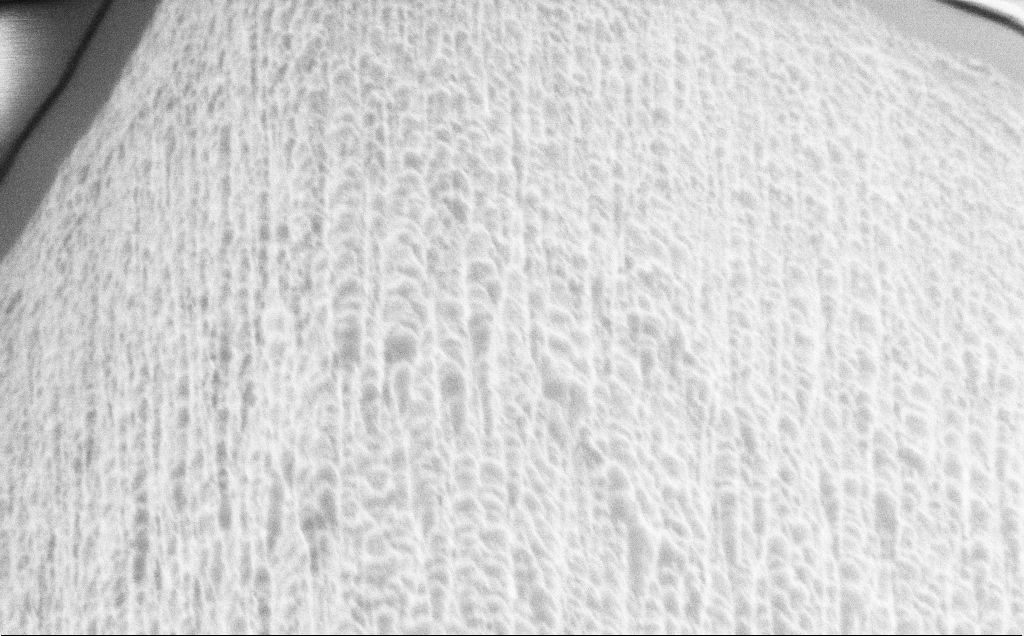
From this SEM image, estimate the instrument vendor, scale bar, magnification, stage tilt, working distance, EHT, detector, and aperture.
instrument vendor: Zeiss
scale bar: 2000 nm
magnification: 23.09 K X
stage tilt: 45°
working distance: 7 mm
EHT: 1.2 kV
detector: InLens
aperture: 30 µm